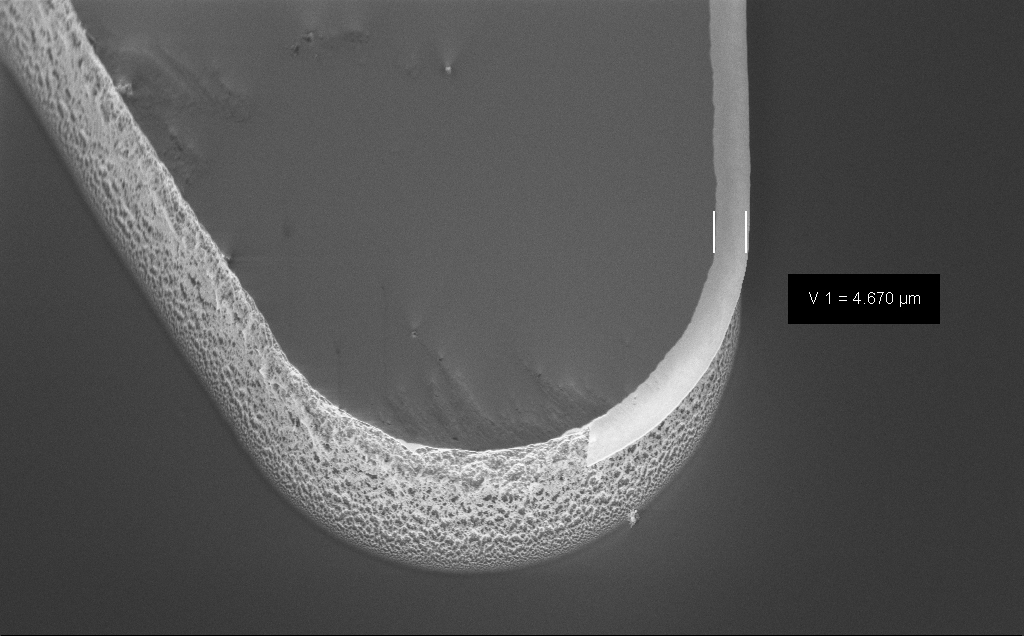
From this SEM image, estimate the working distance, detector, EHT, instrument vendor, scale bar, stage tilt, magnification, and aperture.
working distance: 8 mm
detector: InLens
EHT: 5 kV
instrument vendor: Zeiss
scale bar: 10000 nm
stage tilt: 45°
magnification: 2.52 K X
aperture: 30 µm